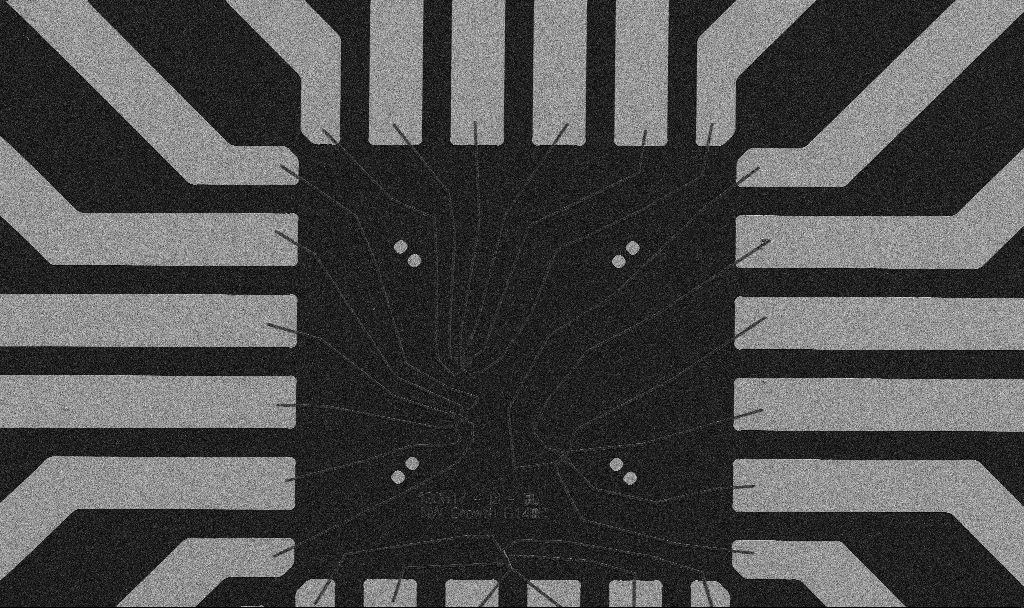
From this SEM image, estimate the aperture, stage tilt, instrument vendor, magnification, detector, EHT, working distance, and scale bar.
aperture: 30 µm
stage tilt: -0°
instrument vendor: Zeiss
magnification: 1 K X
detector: SE2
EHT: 5 kV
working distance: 10.7 mm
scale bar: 20000 nm